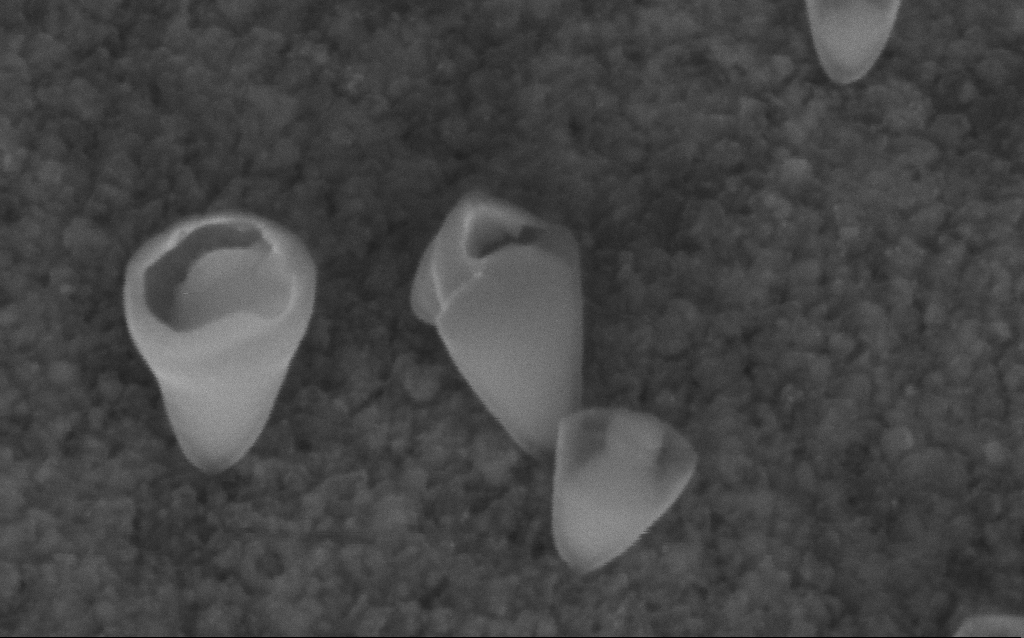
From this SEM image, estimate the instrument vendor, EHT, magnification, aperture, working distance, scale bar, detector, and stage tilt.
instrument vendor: Zeiss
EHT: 5 kV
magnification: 416.83 K X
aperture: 30 µm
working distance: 7 mm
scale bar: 100 nm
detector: InLens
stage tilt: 45°